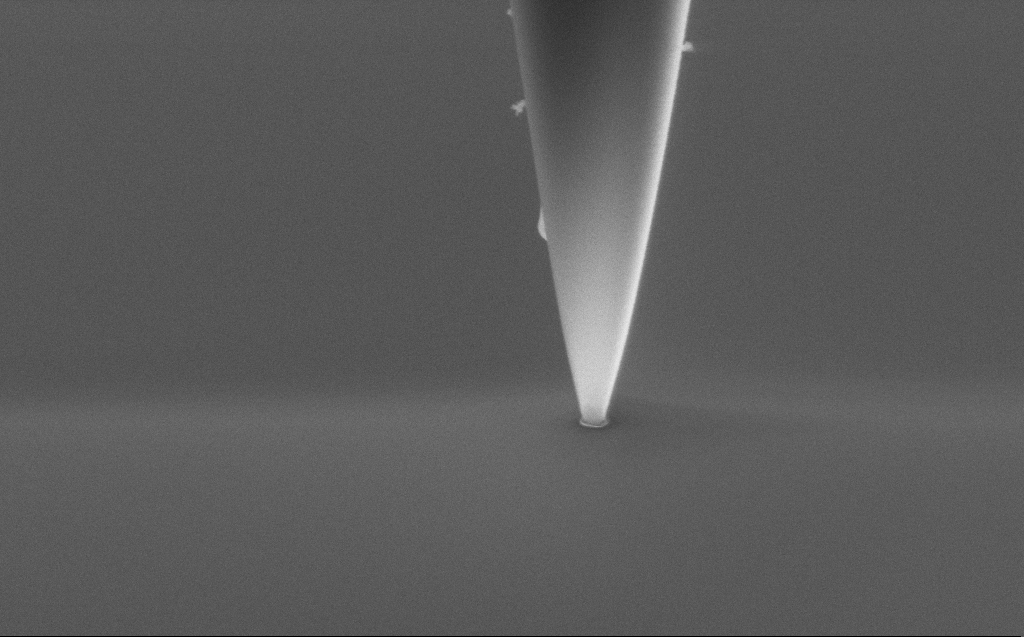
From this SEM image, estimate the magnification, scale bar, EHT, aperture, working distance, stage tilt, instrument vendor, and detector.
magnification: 90.26 K X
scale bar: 200 nm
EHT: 3 kV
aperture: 30 µm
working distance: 3 mm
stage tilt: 45.1°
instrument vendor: Zeiss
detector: SE2